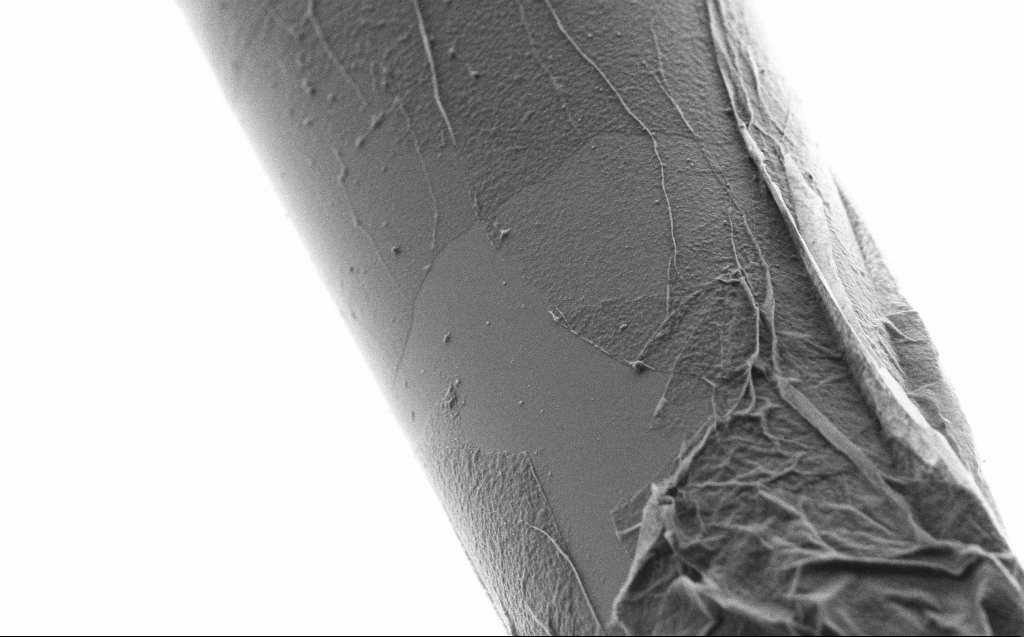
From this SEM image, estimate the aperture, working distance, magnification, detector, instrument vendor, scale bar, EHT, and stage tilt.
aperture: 30 µm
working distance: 3 mm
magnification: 10 K X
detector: SE2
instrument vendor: Zeiss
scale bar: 2000 nm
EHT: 2 kV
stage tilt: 45°